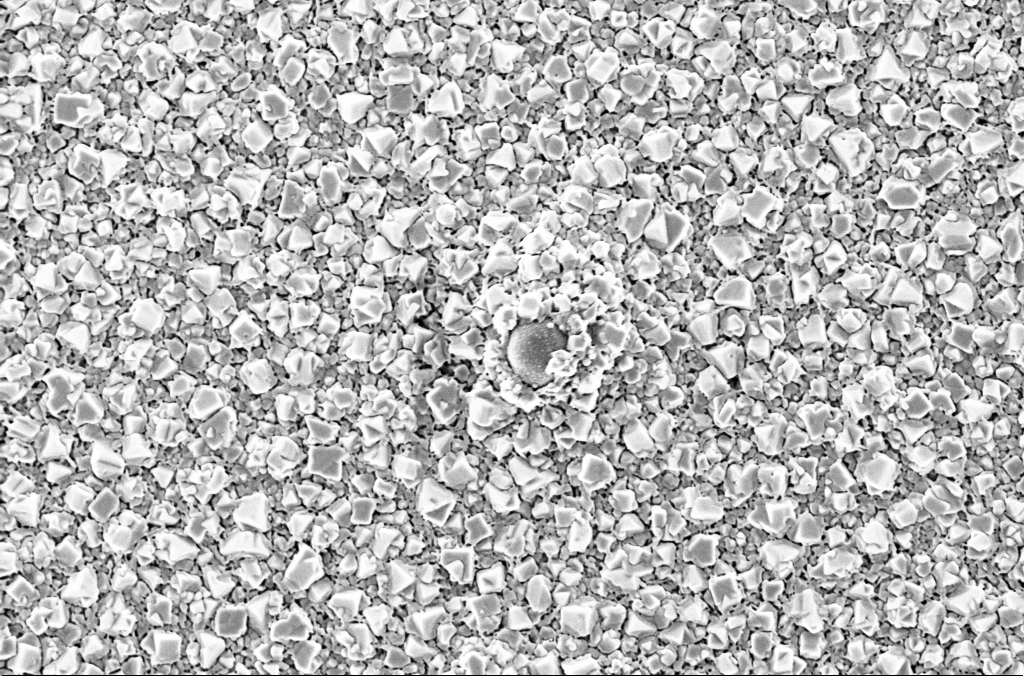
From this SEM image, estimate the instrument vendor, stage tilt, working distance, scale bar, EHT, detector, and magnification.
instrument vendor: Zeiss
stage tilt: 0°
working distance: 1.9 mm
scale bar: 2000 nm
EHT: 2 kV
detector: InLens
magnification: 30 K X